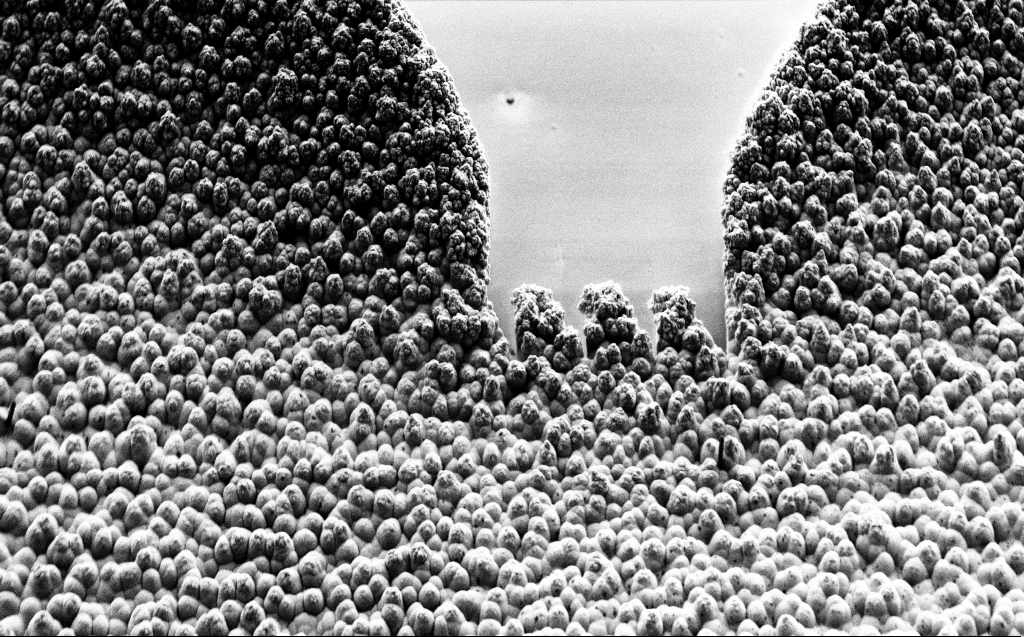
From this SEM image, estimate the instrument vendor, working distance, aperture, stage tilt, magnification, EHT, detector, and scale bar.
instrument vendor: Zeiss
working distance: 7 mm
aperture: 30 µm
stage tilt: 45°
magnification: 2.2 K X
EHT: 1 kV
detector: SE2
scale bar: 20000 nm